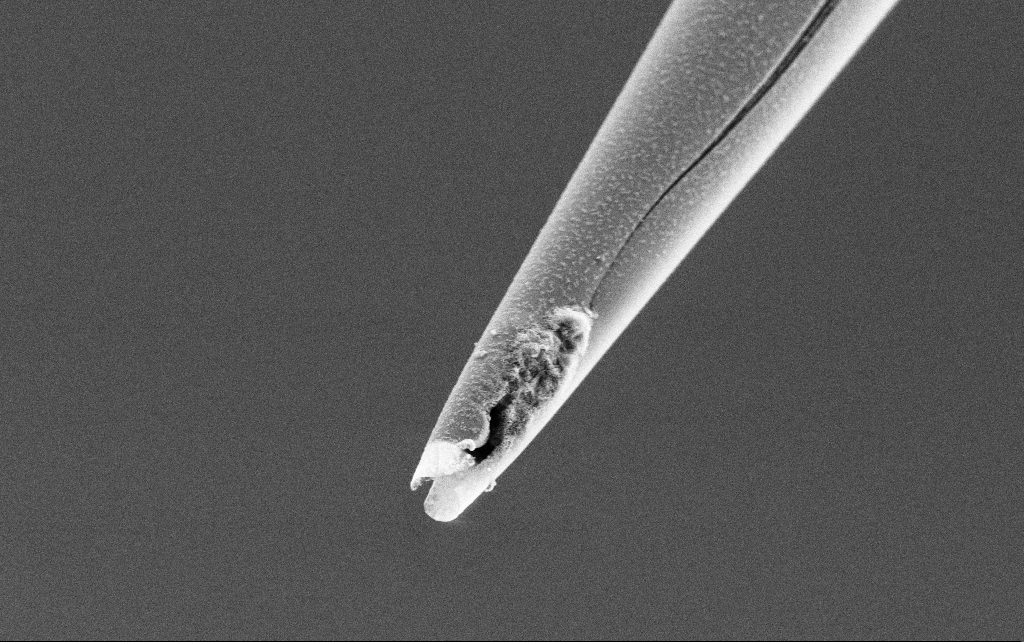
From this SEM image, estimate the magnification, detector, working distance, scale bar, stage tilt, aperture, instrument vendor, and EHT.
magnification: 15 K X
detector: SE2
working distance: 7.5 mm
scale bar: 1000 nm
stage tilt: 45°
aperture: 30 µm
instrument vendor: Zeiss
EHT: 3 kV